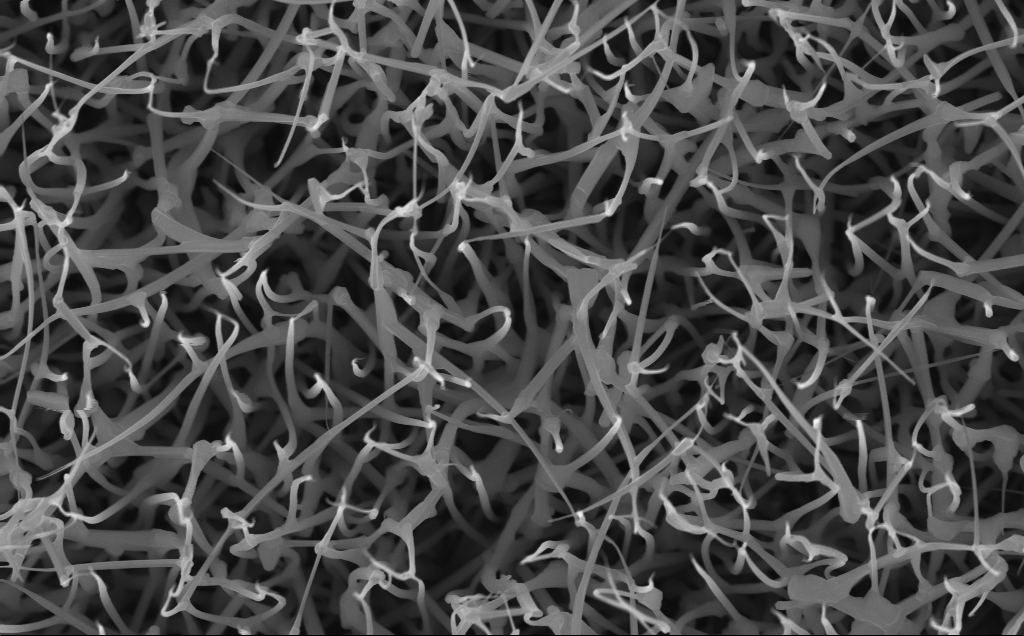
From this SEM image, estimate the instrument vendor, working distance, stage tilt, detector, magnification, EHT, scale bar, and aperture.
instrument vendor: Zeiss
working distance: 5 mm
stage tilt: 0°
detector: InLens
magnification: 40 K X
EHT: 10 kV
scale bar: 1000 nm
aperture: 30 µm